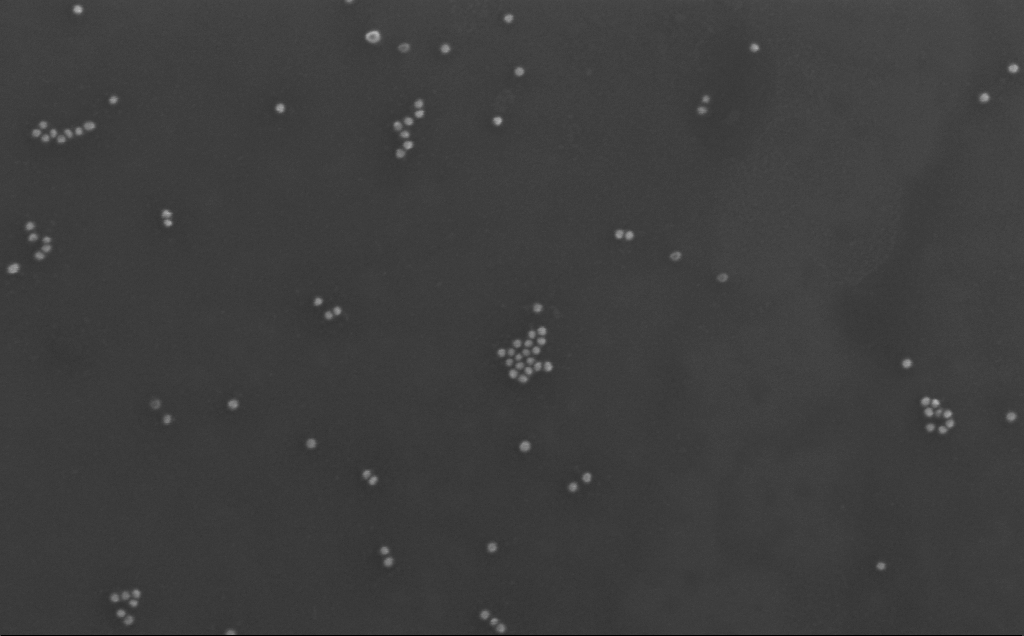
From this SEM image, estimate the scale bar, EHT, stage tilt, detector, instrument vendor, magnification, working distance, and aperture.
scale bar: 200 nm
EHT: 10 kV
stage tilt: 0°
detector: InLens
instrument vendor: Zeiss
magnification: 196.58 K X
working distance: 3 mm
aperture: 30 µm